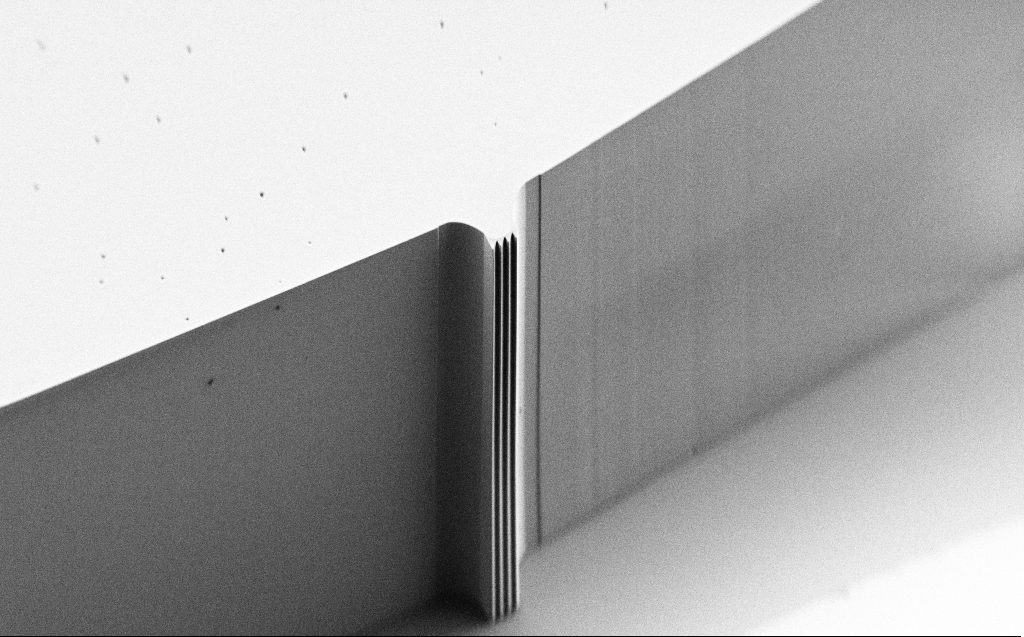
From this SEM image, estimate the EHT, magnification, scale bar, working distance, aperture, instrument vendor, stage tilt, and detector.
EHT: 0.9 kV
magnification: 0.28 K X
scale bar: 100000 nm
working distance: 5 mm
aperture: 30 µm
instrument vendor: Zeiss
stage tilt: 45°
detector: SE2